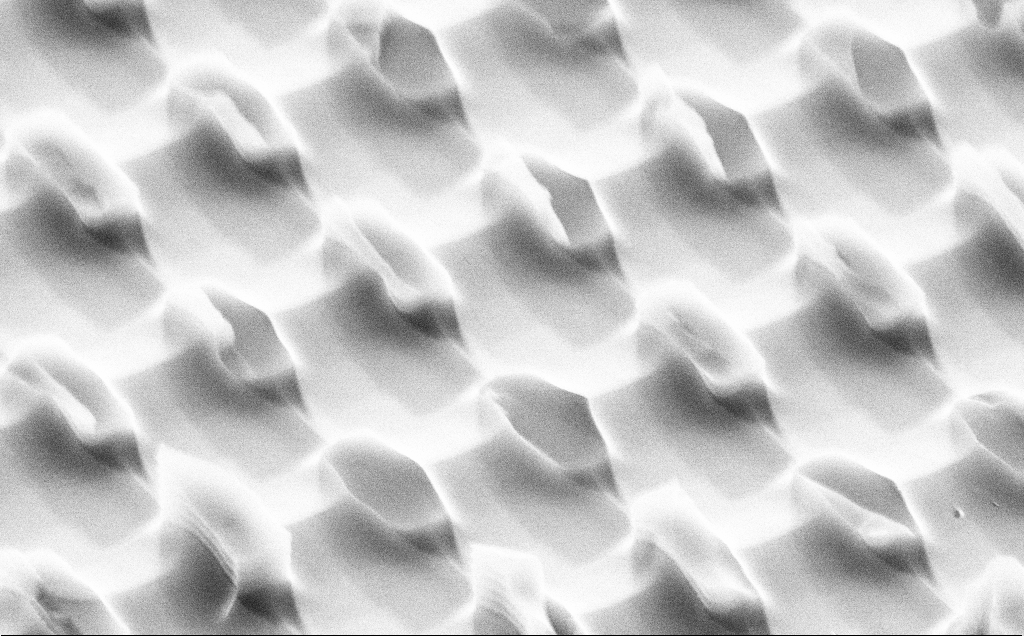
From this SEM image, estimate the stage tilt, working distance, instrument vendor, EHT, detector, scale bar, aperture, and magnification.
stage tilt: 51.2°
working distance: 9 mm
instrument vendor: Zeiss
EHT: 10 kV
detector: SE2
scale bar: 2000 nm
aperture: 30 µm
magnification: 17.55 K X